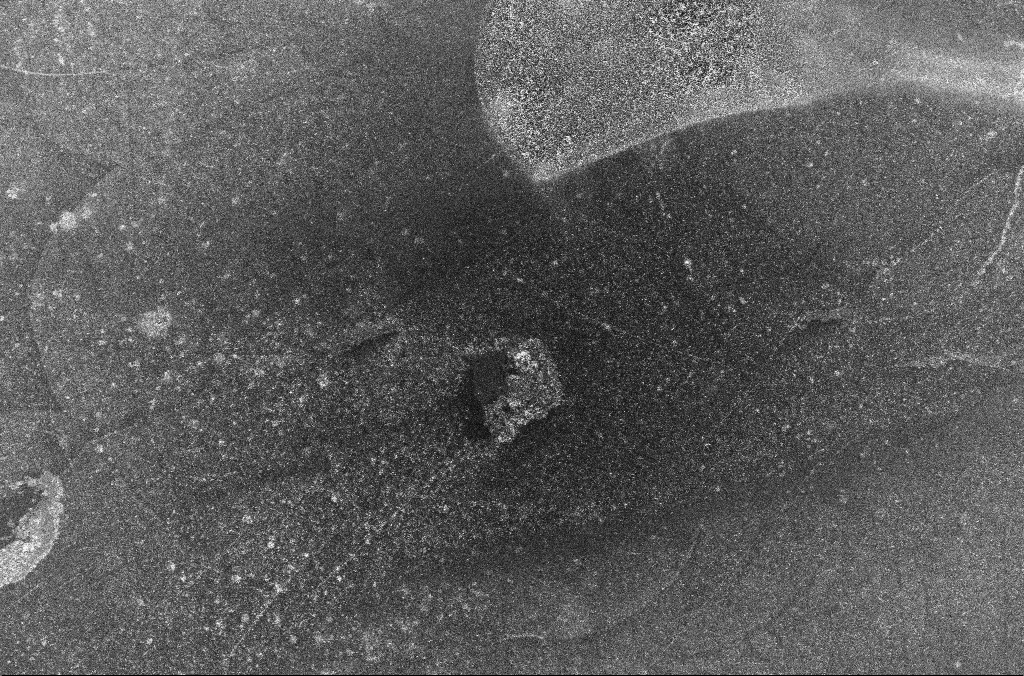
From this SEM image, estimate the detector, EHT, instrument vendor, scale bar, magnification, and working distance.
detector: InLens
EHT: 10 kV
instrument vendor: Zeiss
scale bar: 100000 nm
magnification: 0.5 K X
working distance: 3.3 mm